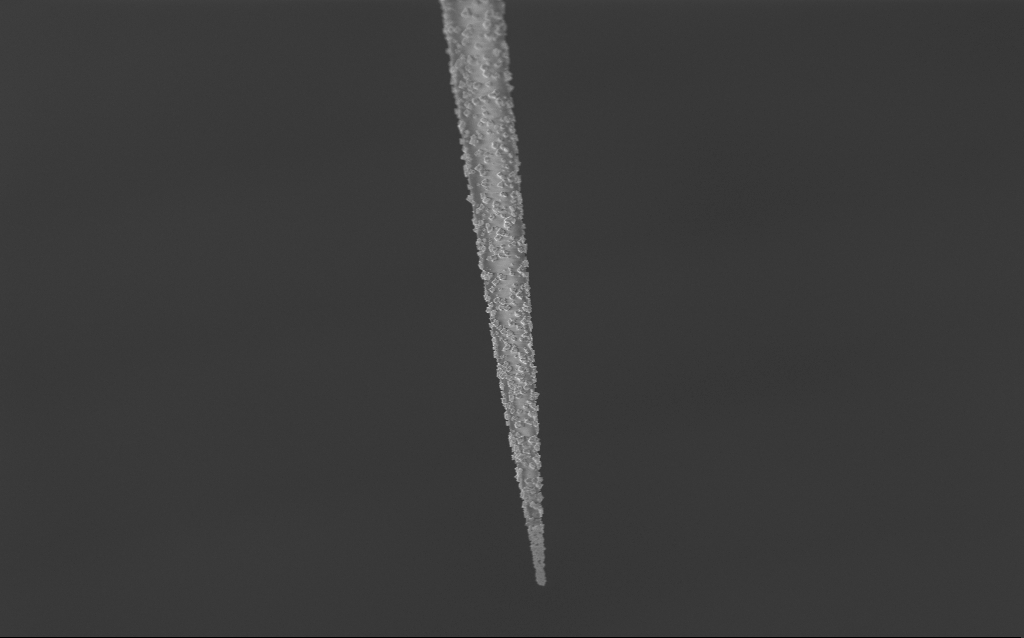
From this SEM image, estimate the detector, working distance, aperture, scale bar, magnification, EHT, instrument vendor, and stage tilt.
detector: InLens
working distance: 7.7 mm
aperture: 30 µm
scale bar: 20000 nm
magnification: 1 K X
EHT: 1.5 kV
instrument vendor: Zeiss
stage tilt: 45°